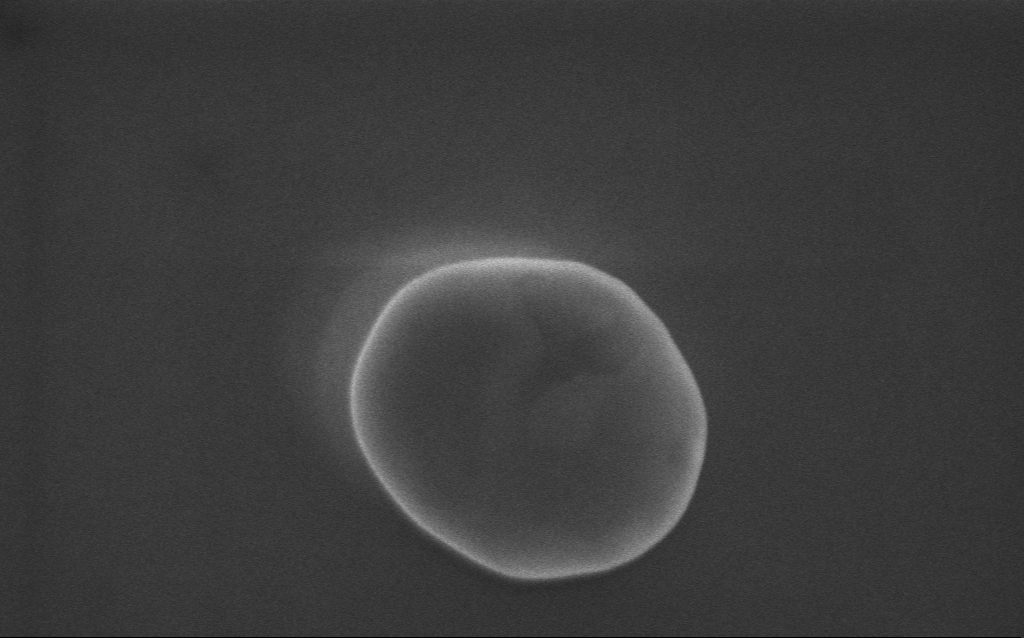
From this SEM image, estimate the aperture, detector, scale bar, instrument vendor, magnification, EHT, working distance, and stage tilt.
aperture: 30 µm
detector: InLens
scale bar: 200 nm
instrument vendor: Zeiss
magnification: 141 K X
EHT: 5 kV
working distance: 3 mm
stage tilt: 0°